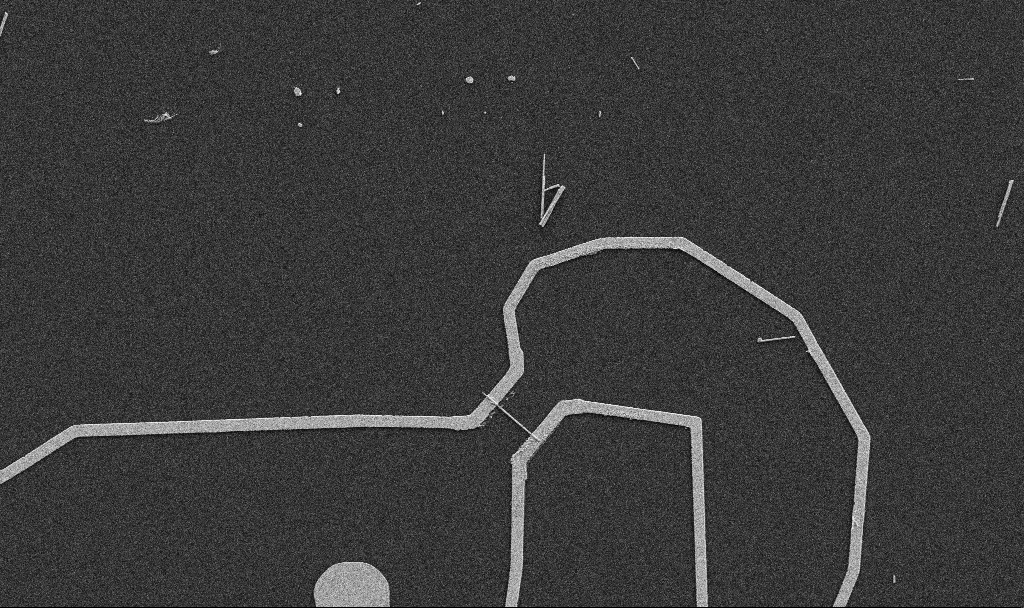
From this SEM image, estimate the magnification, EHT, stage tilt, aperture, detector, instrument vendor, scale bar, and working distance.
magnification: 5 K X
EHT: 5 kV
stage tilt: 0°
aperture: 30 µm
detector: SE2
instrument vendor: Zeiss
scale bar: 10000 nm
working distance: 10.7 mm